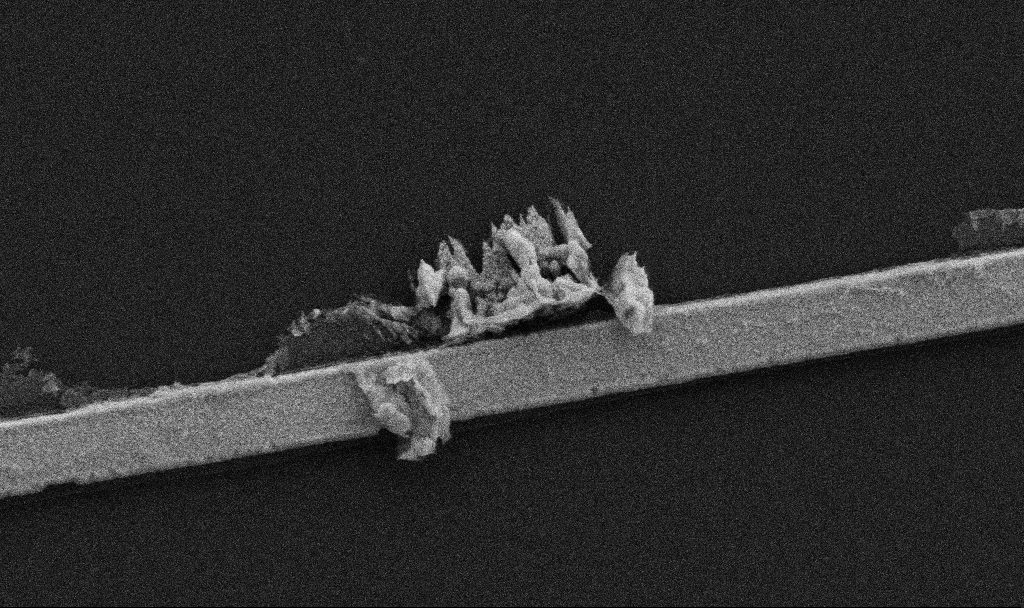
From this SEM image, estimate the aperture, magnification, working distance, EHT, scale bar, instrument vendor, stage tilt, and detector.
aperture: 30 µm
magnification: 32.83 K X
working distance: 10.7 mm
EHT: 5 kV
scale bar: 1000 nm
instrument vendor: Zeiss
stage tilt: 0°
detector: SE2